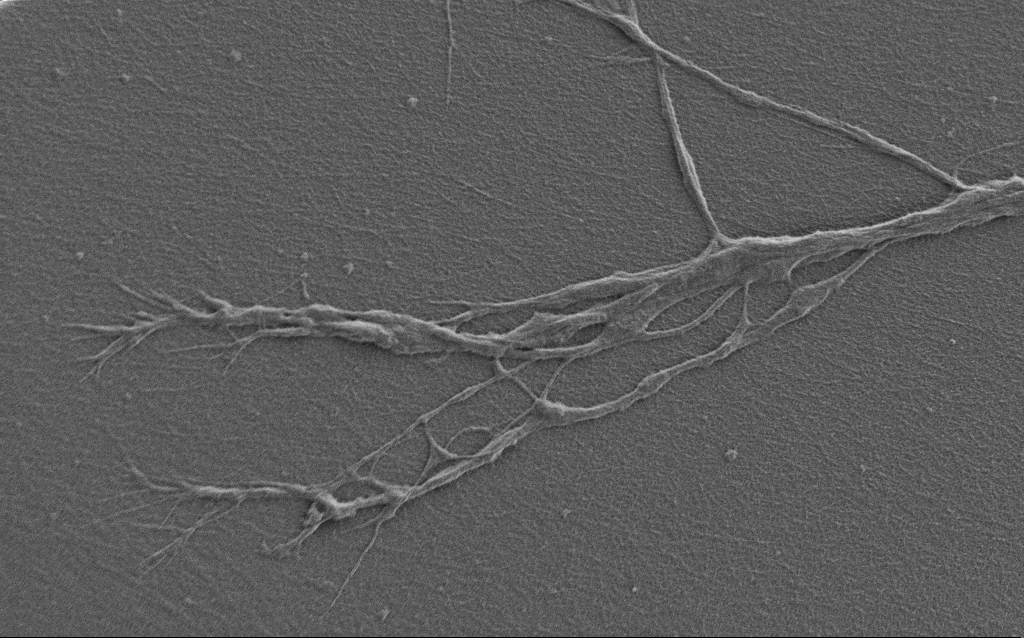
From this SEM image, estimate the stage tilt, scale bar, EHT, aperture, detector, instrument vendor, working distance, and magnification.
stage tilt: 0°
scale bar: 10000 nm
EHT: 0.9 kV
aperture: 30 µm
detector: SE2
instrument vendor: Zeiss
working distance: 7 mm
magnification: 6 K X